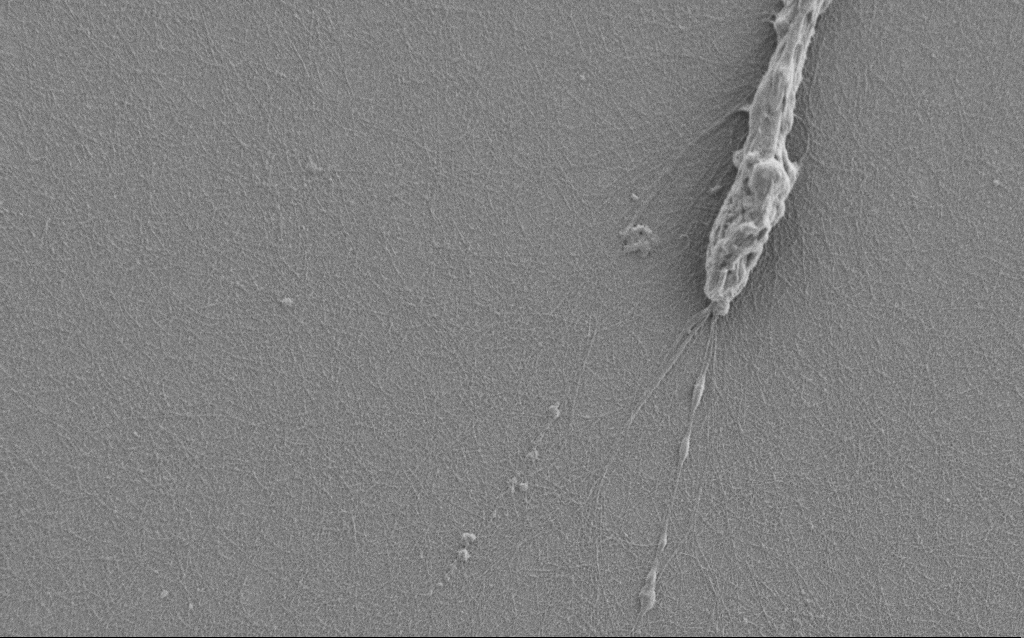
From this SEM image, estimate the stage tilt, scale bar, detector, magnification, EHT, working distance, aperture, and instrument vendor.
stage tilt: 0°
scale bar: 2000 nm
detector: SE2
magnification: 7.5 K X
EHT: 1 kV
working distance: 6 mm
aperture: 30 µm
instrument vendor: Zeiss